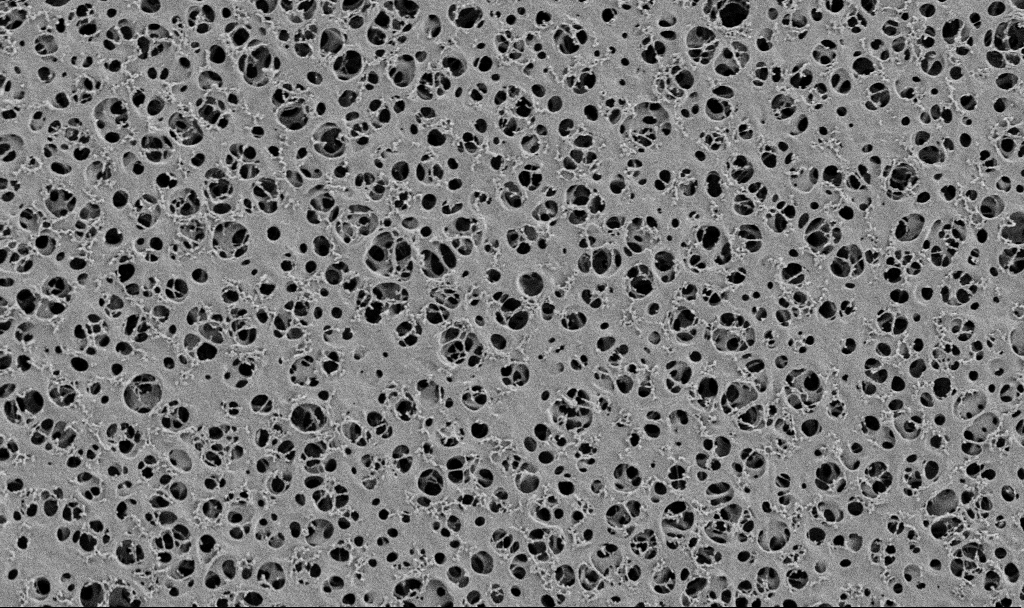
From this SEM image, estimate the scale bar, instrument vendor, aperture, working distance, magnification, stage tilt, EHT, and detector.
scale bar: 10000 nm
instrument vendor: Zeiss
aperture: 30 µm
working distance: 3.7 mm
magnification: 5 K X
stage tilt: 0°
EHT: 2 kV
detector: SE2